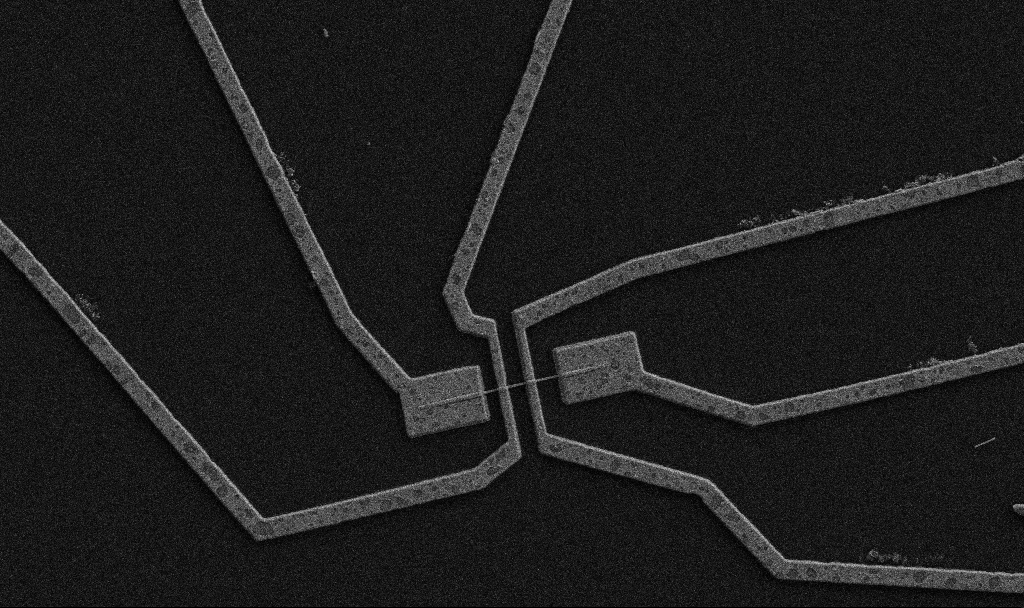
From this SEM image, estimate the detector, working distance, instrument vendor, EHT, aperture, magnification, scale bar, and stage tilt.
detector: SE2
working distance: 9.7 mm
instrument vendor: Zeiss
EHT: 5 kV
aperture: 30 µm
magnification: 10 K X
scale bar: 2000 nm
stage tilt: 0°